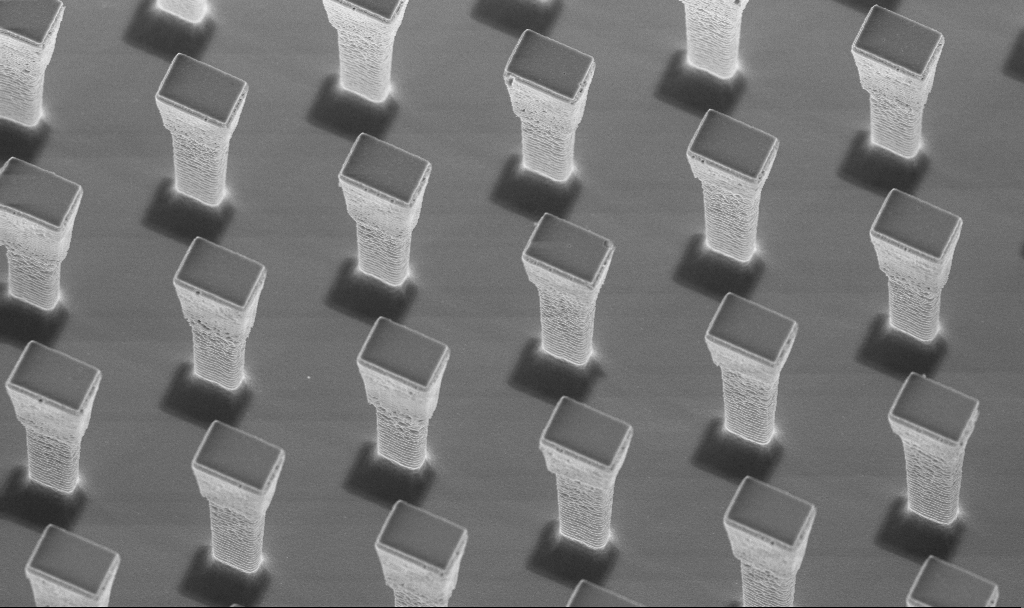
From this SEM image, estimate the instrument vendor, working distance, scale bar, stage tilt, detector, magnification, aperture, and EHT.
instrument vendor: Zeiss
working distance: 4.3 mm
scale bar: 10000 nm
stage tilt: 20°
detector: InLens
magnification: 6.2 K X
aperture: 30 µm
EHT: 5 kV